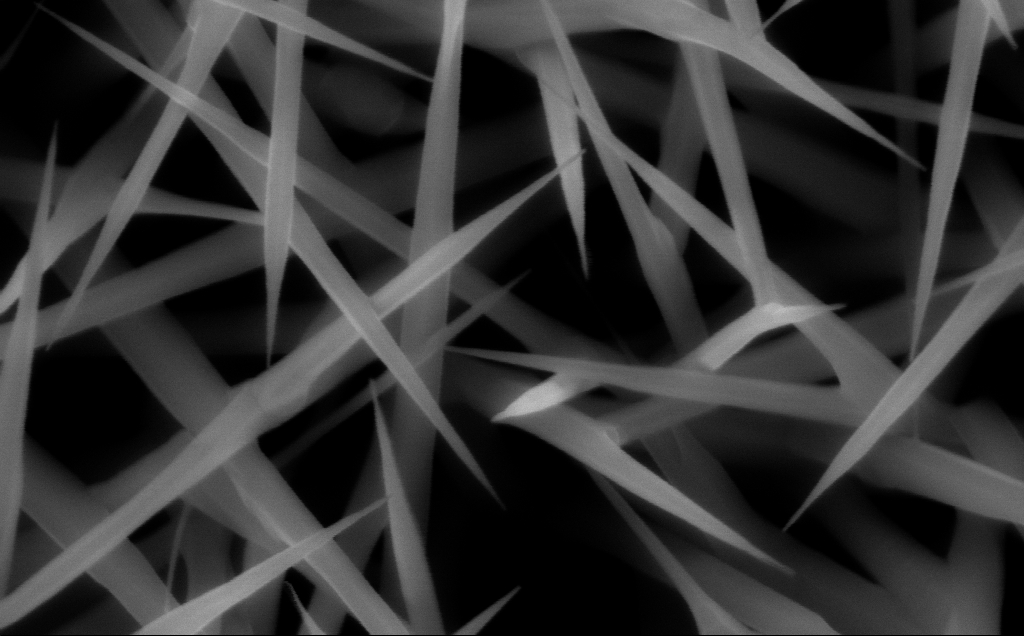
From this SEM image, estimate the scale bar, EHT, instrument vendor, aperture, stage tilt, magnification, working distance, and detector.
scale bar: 100 nm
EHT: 10 kV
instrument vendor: Zeiss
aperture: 30 µm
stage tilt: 0°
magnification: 150 K X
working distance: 7 mm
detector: InLens